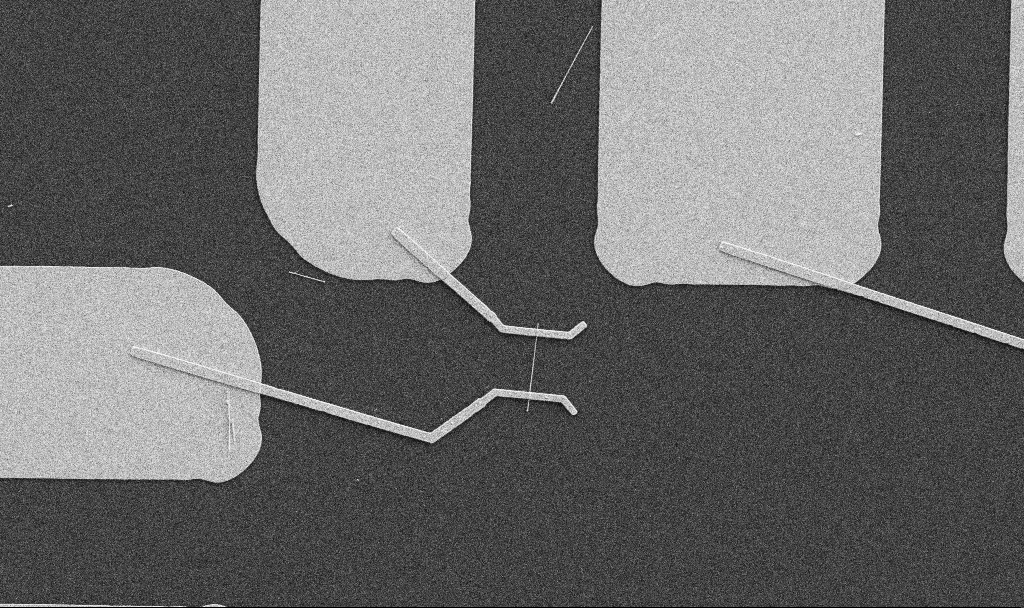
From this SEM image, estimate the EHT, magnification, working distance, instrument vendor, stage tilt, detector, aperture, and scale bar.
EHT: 5 kV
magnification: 5 K X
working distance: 10.7 mm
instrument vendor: Zeiss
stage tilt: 0°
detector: SE2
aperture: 30 µm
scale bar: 10000 nm